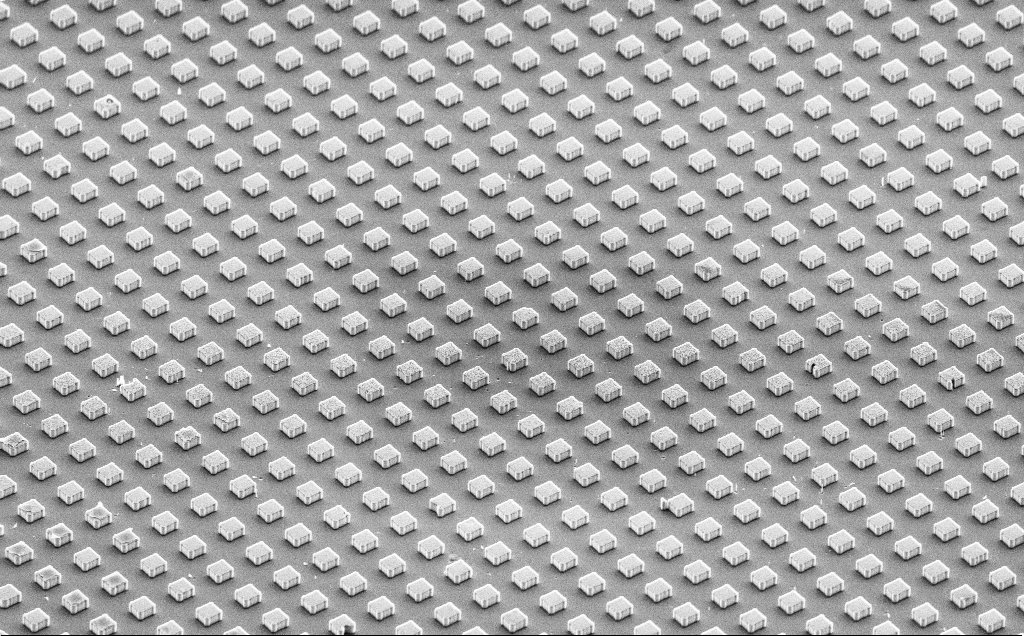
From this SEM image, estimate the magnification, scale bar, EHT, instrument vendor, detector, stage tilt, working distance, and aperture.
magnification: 0.874 K X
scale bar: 20000 nm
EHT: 10 kV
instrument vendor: Zeiss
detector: SE2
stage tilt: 50.6°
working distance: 13 mm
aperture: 30 µm